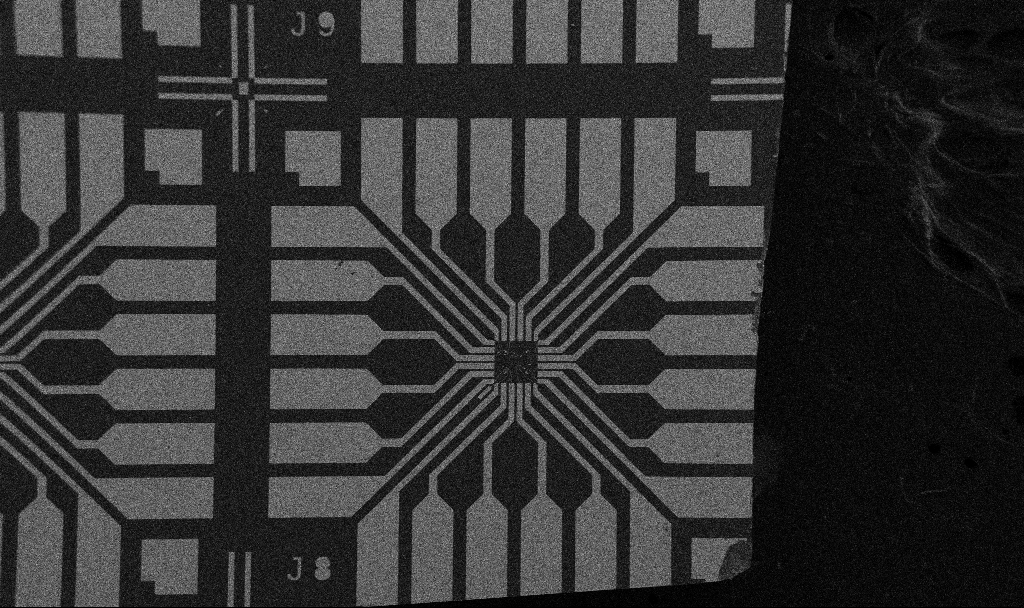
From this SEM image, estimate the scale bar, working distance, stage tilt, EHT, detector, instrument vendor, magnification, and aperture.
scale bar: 200000 nm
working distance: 10.7 mm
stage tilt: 0°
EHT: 5 kV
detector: SE2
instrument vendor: Zeiss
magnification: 0.1 K X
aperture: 30 µm